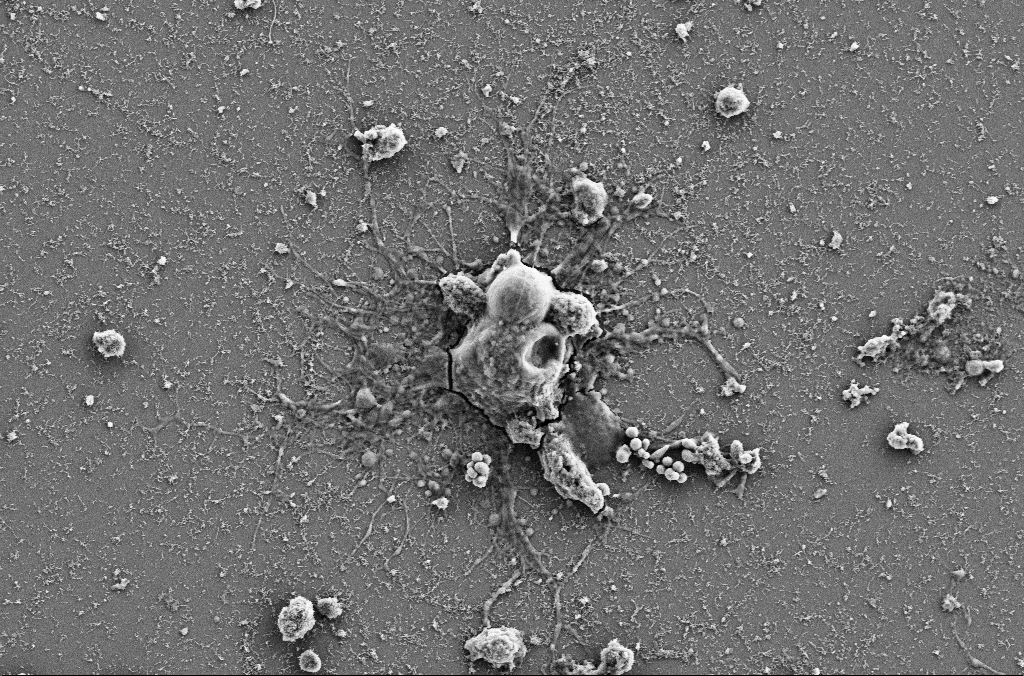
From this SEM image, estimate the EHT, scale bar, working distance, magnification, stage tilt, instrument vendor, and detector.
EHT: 5 kV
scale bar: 10000 nm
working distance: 4 mm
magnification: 4 K X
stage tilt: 0°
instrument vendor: Zeiss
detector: SE2